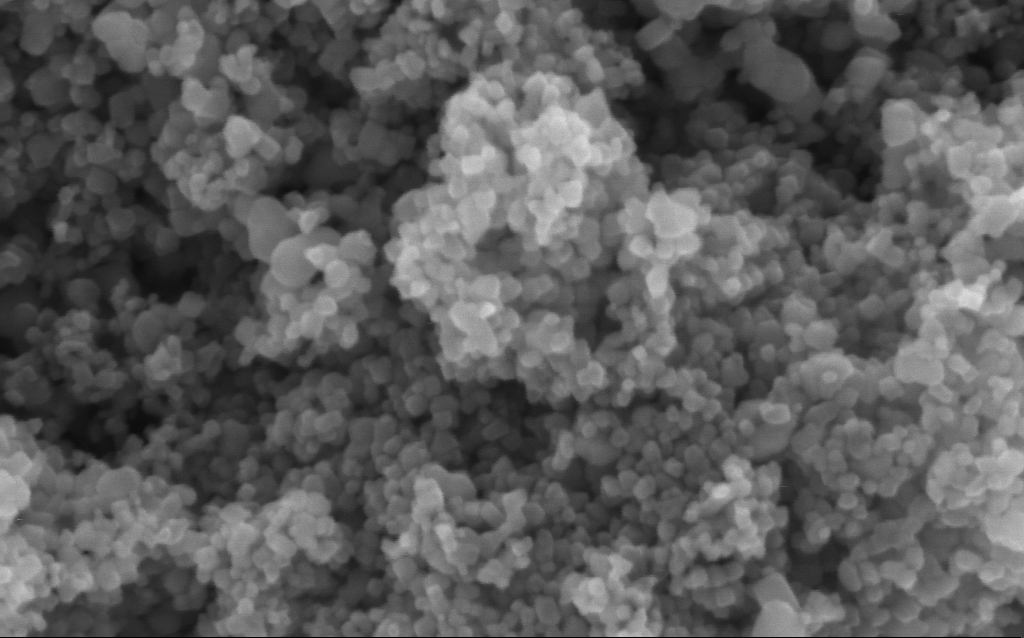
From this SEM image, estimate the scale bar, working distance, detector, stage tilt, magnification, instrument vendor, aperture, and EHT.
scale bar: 100 nm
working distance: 4.2 mm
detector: InLens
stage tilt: -0°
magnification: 294.05 K X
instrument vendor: Zeiss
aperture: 30 µm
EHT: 5 kV